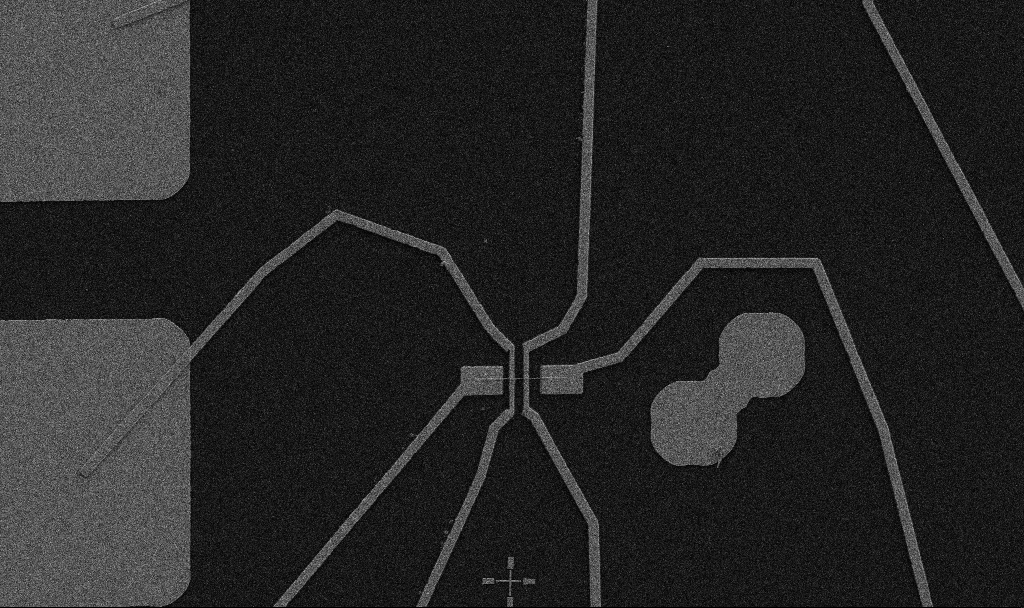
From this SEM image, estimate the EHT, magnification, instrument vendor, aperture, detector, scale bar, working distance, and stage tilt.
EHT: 5 kV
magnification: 5 K X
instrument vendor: Zeiss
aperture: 30 µm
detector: SE2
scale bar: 10000 nm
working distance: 10.7 mm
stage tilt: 0°